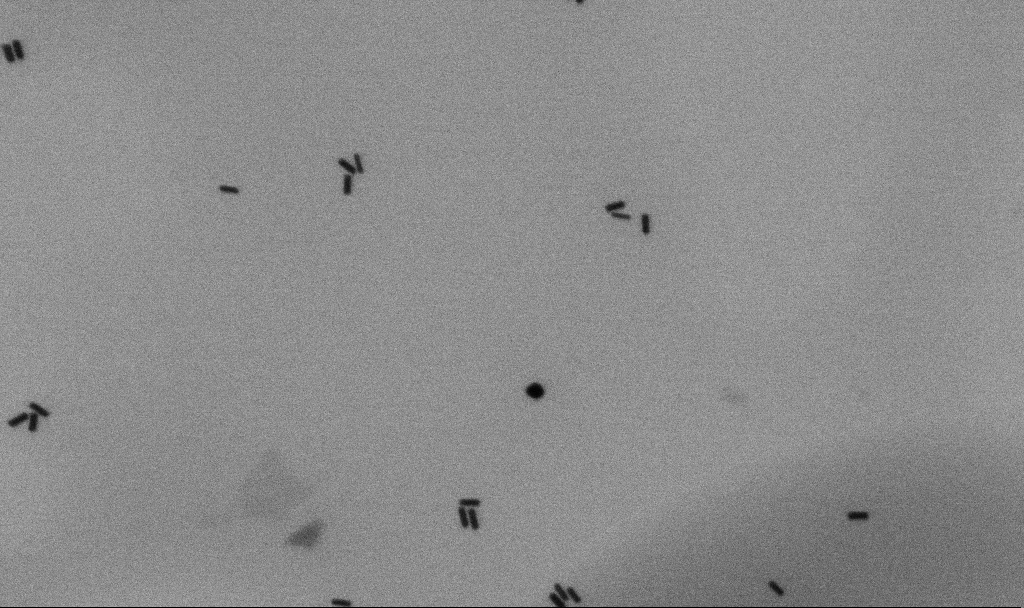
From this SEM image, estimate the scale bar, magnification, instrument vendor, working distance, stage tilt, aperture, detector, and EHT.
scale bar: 200 nm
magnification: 100 K X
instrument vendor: Zeiss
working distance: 4.5 mm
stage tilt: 0°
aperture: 30 µm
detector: SE2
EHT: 5 kV